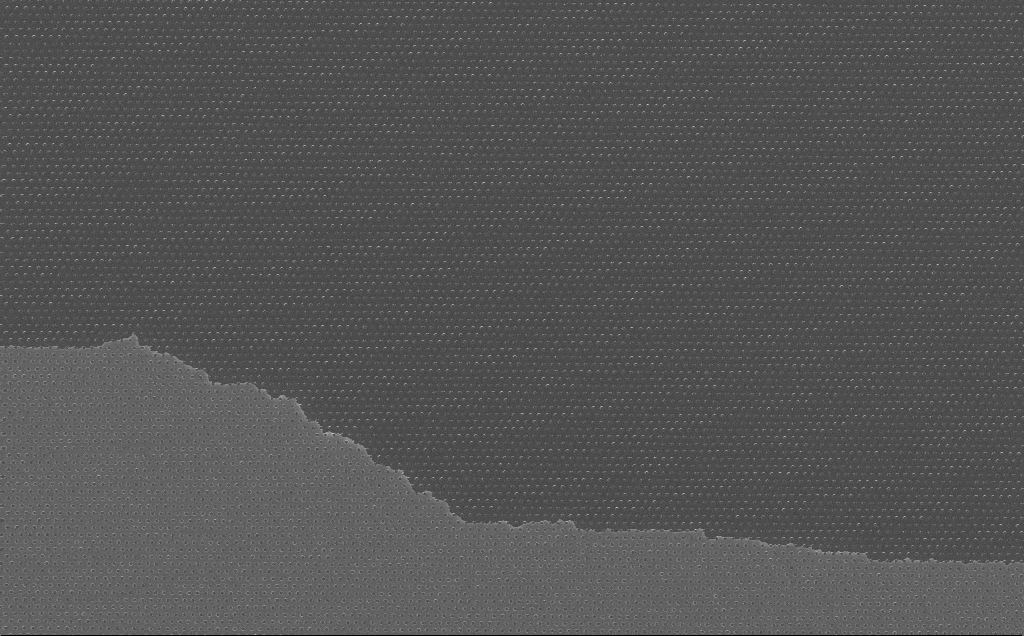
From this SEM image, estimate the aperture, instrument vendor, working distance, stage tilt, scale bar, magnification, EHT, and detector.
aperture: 30 µm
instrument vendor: Zeiss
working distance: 7 mm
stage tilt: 0°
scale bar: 2000 nm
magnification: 7.71 K X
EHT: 10 kV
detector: InLens